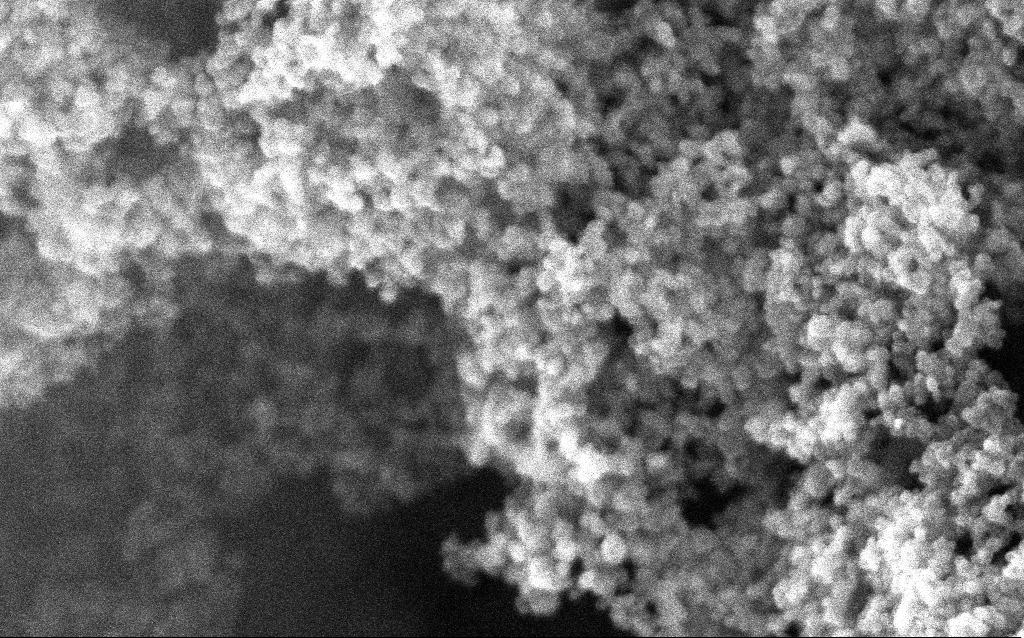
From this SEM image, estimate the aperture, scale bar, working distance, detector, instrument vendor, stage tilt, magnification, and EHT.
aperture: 30 µm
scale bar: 100 nm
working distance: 2.4 mm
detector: InLens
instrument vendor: Zeiss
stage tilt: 0°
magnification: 204.13 K X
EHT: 10 kV